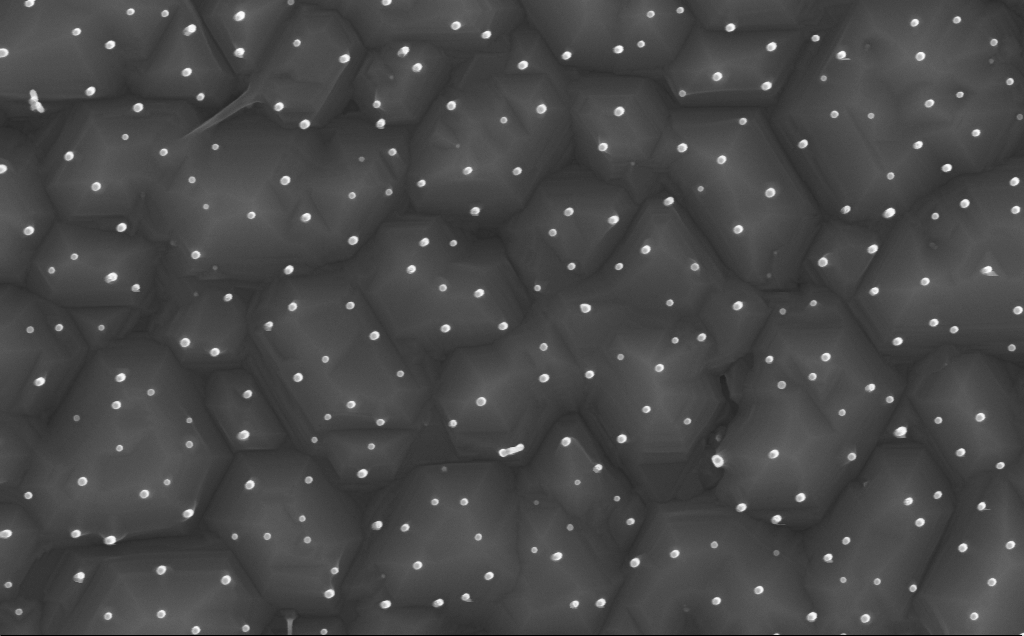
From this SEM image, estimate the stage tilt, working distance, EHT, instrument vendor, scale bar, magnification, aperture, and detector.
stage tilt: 0.2°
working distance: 7 mm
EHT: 10 kV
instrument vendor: Zeiss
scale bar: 200 nm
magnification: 80 K X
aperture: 30 µm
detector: InLens